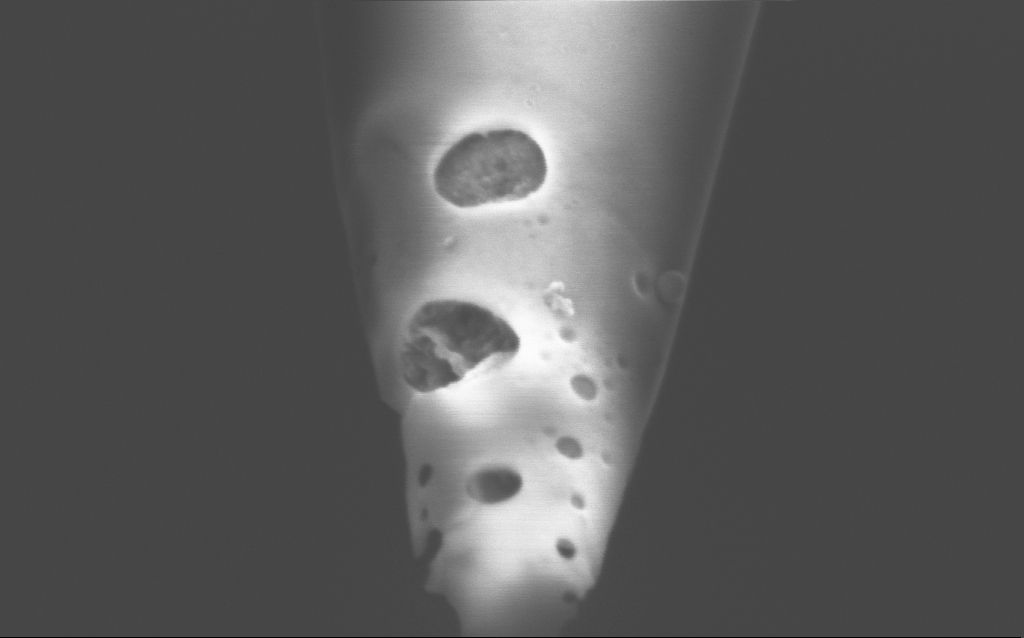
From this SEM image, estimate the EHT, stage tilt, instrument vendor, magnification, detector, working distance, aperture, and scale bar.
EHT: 2 kV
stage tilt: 45°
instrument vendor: Zeiss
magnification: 200 K X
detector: InLens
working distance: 6 mm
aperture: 30 µm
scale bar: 200 nm